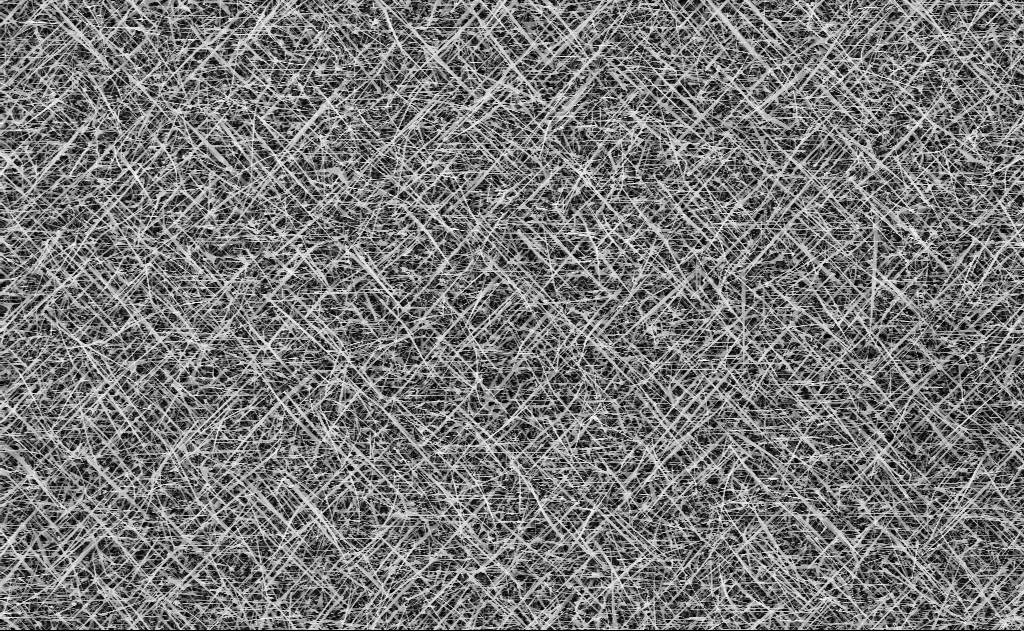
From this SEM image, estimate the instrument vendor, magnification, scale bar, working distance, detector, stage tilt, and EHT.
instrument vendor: Zeiss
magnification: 5 K X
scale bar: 10000 nm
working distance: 11 mm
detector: InLens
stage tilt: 0°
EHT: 10 kV